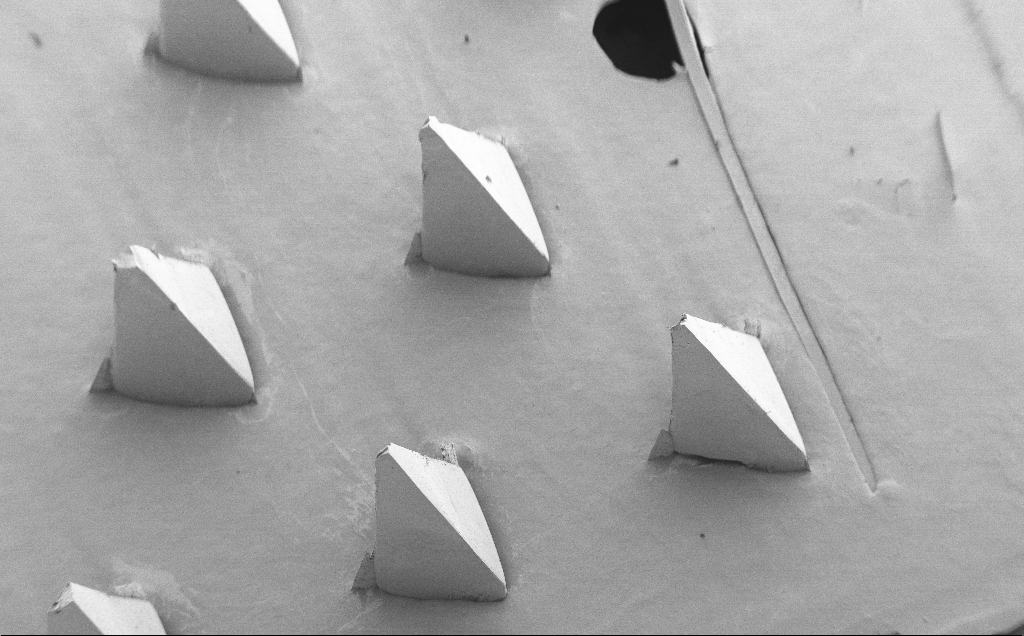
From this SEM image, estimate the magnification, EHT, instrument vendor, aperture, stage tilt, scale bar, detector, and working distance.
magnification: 0.079 K X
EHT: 5 kV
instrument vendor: Zeiss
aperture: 30 µm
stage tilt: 40°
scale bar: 200000 nm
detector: SE2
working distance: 8 mm